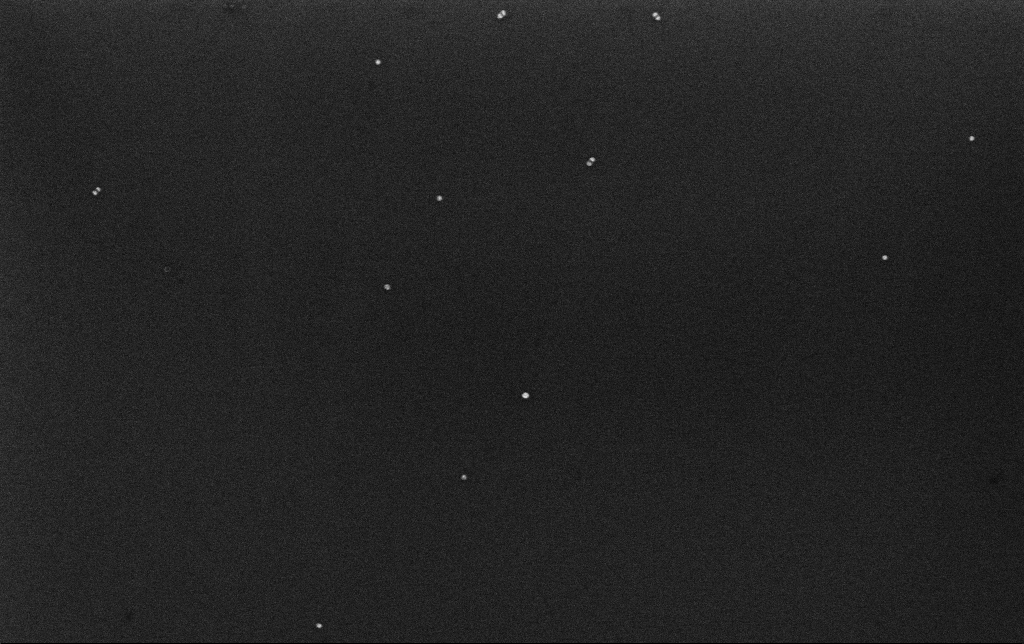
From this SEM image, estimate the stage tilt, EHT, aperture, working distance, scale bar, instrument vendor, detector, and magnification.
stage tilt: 0°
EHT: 10 kV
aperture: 30 µm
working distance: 3.2 mm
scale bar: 200 nm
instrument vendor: Zeiss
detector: InLens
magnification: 100 K X